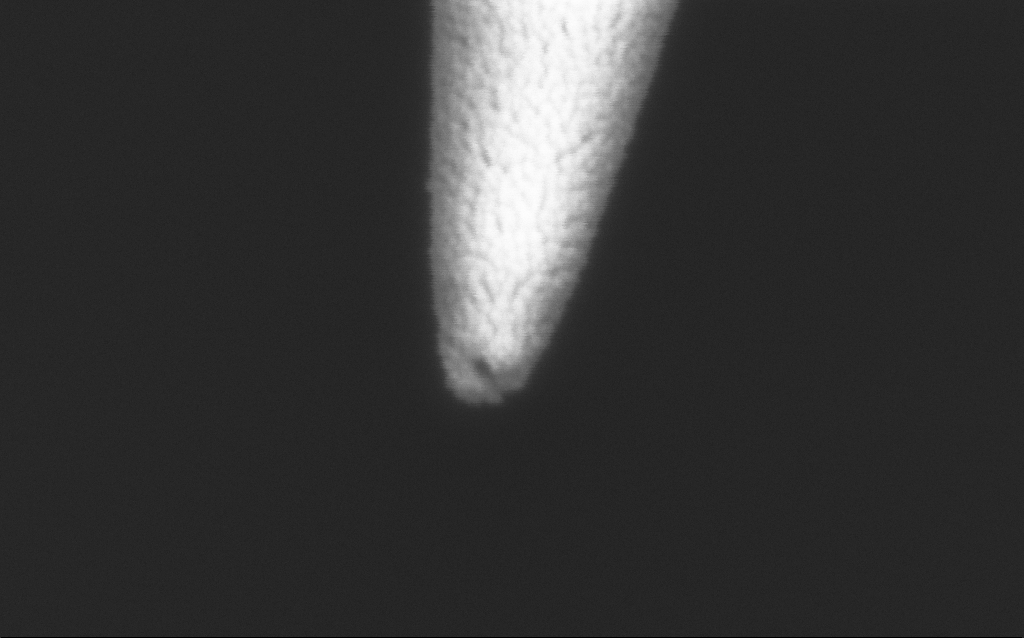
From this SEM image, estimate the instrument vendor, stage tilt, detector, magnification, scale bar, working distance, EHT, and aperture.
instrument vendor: Zeiss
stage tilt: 45°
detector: InLens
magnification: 300 K X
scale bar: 200 nm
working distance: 7.6 mm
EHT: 2 kV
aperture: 30 µm